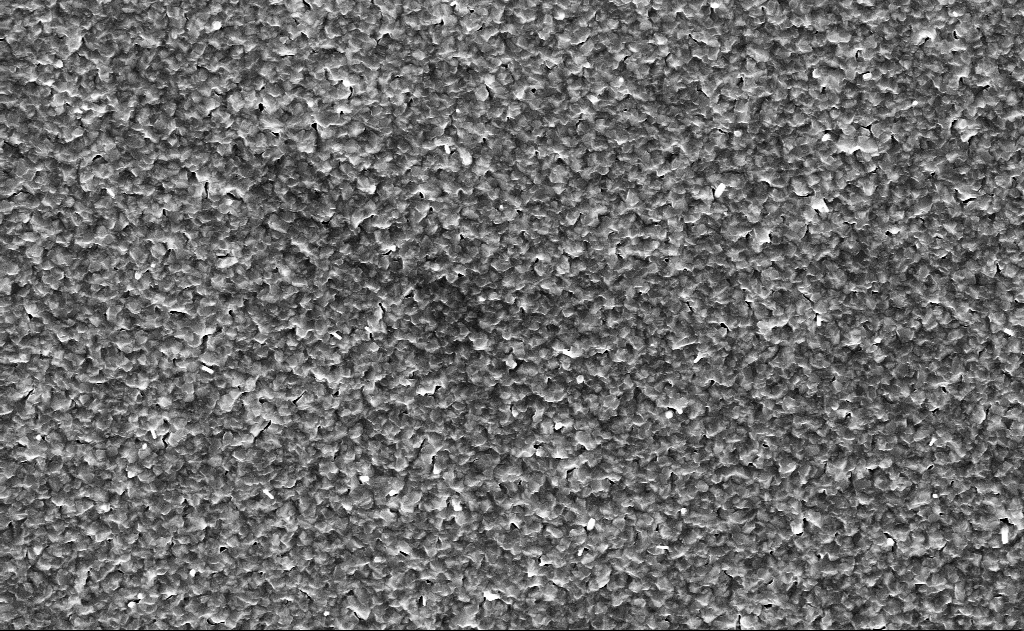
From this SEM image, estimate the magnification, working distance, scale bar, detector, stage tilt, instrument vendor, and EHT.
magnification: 20 K X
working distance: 11 mm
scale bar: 1000 nm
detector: InLens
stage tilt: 0°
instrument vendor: Zeiss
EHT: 10 kV